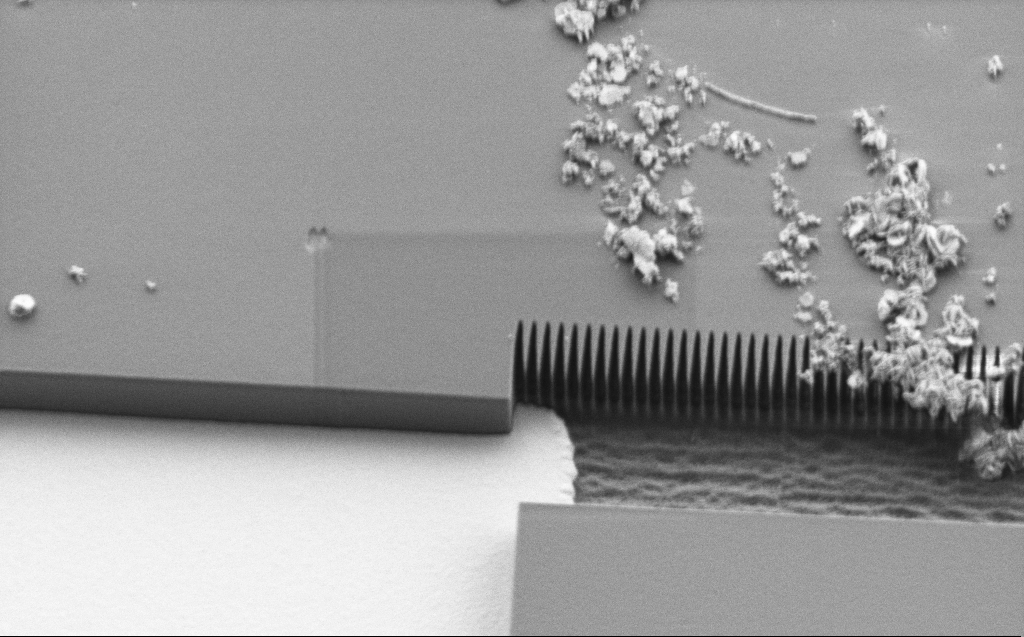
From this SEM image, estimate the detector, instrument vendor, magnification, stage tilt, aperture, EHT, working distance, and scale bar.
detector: SE2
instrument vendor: Zeiss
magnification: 10.05 K X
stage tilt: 30°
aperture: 30 µm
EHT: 1.1 kV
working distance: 6 mm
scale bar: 2000 nm